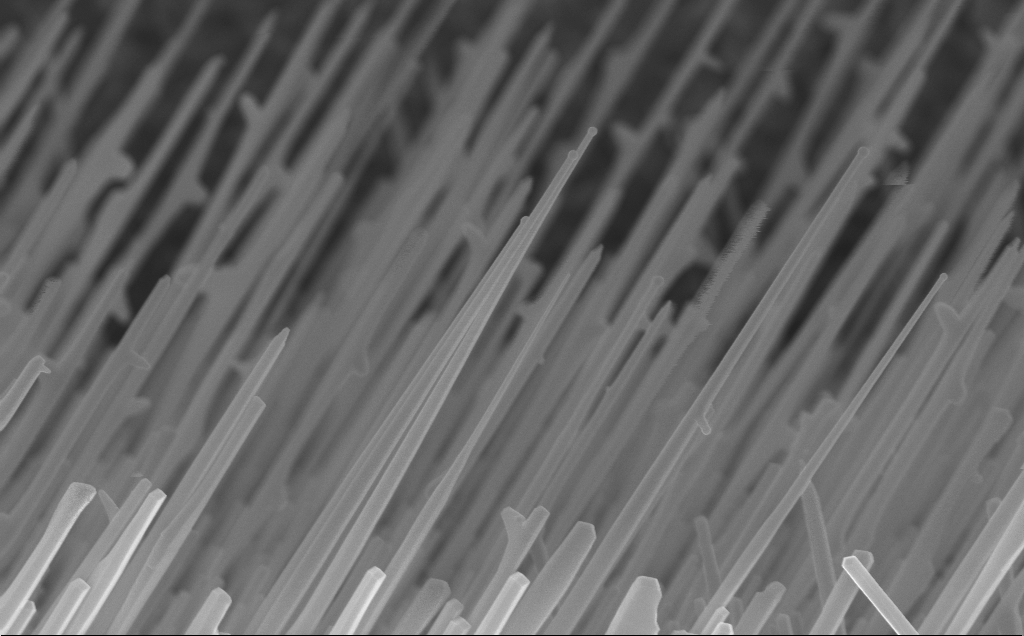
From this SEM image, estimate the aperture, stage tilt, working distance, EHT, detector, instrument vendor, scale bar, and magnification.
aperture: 30 µm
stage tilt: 0°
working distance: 7 mm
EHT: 10 kV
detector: InLens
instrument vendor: Zeiss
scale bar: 1000 nm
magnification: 37.3 K X